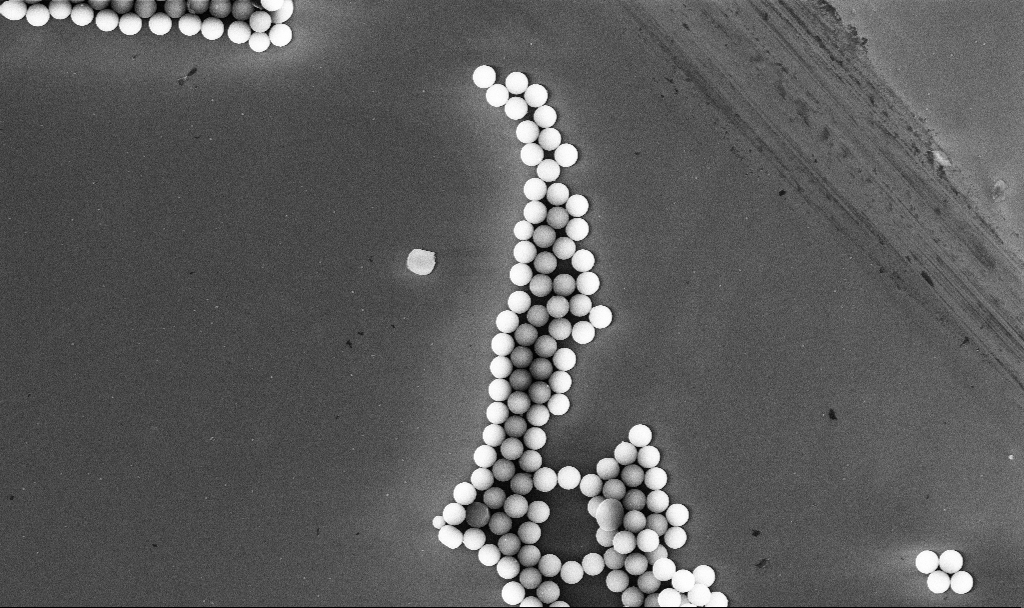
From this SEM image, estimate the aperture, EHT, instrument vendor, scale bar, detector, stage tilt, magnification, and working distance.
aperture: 30 µm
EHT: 10 kV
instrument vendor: Zeiss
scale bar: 10000 nm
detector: InLens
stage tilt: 0°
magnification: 4.07 K X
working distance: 5.2 mm